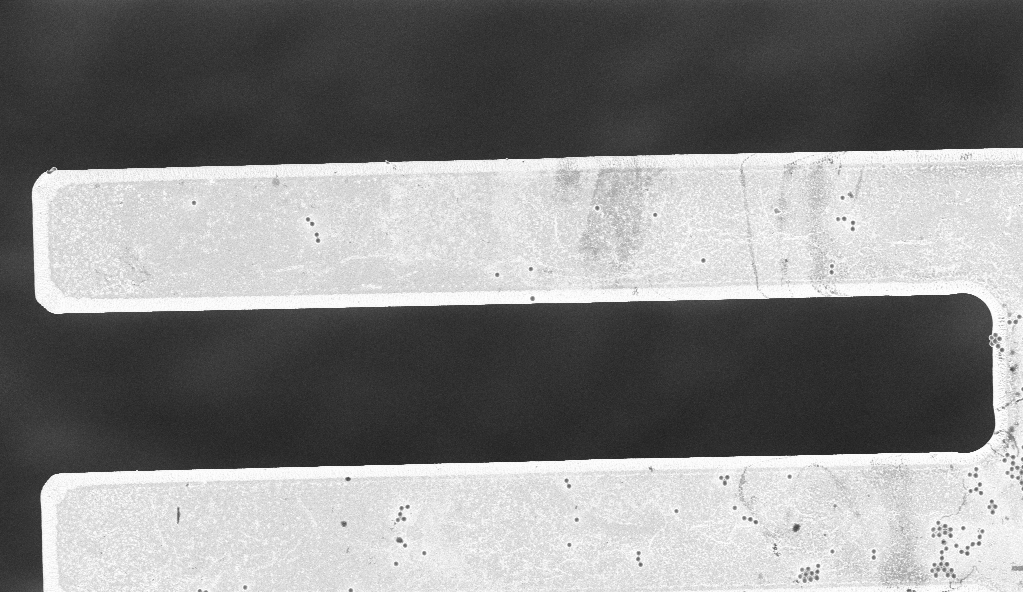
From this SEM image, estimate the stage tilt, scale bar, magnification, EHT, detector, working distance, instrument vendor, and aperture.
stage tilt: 0°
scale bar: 20000 nm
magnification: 2.8 K X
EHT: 3 kV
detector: InLens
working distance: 7 mm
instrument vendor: Zeiss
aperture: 30 µm